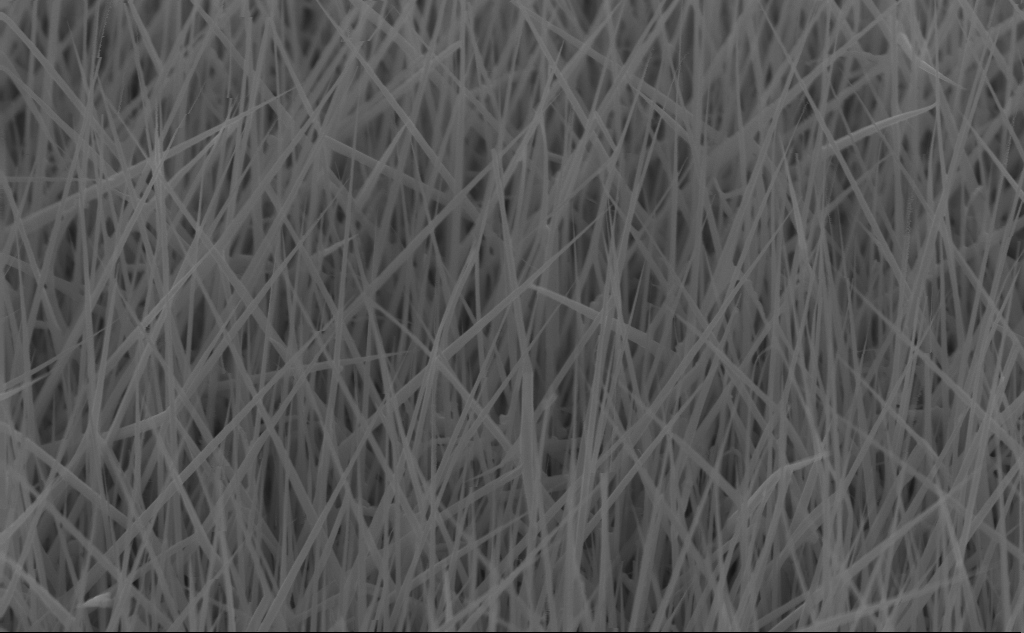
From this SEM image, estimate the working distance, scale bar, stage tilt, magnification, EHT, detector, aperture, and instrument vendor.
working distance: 4 mm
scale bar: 1000 nm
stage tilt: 45°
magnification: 40 K X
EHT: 10 kV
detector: InLens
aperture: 30 µm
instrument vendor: Zeiss